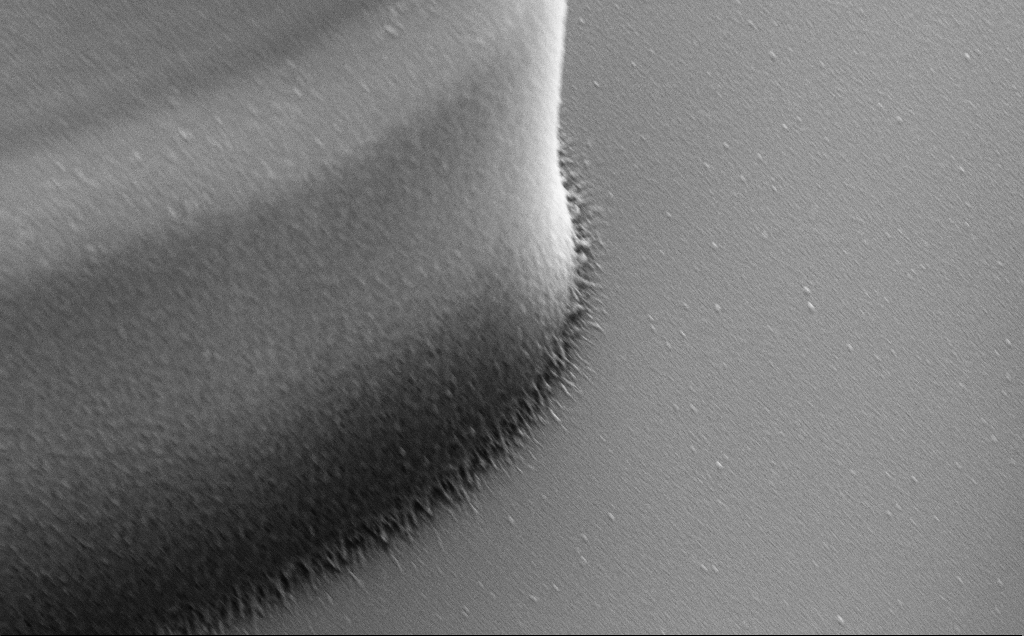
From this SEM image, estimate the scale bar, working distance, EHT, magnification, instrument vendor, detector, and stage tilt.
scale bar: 1000 nm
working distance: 11 mm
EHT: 10 kV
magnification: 17.93 K X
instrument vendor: Zeiss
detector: SE2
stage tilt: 35.3°